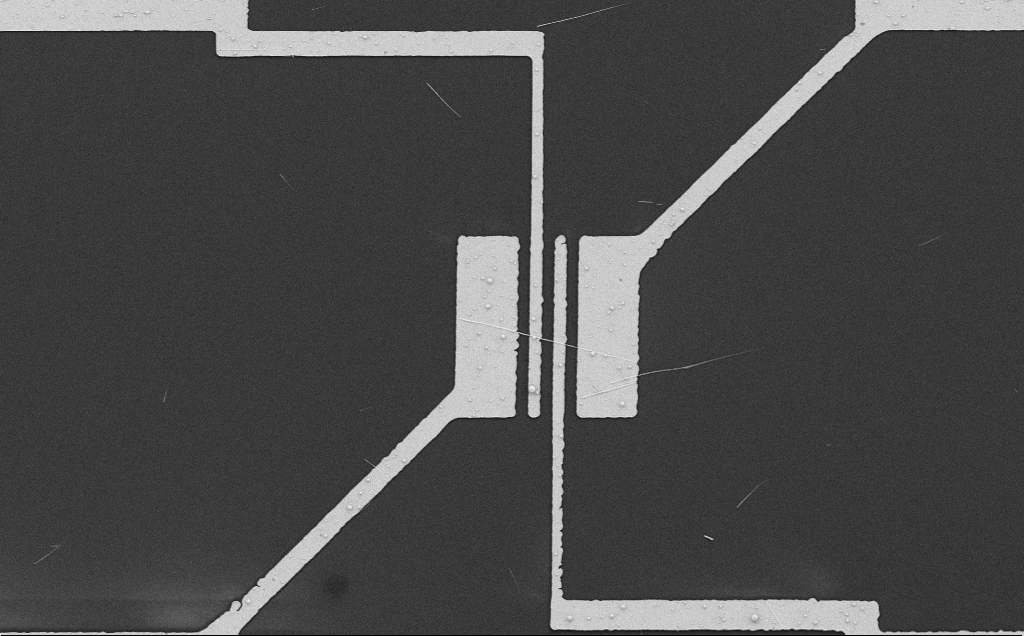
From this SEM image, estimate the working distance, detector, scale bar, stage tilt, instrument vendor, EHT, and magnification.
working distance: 8 mm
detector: SE2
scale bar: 20000 nm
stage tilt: -0.7°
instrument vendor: Zeiss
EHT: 5 kV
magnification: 2.25 K X